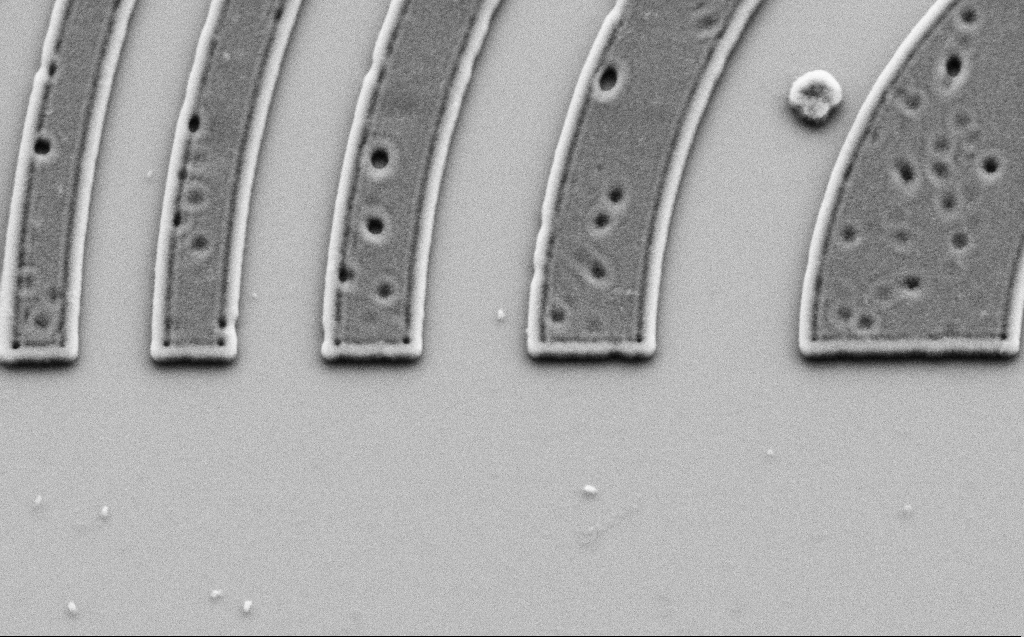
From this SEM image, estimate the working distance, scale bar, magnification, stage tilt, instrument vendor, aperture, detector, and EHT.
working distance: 5 mm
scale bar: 1000 nm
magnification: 29.24 K X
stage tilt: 30°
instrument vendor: Zeiss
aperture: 30 µm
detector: SE2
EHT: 3 kV